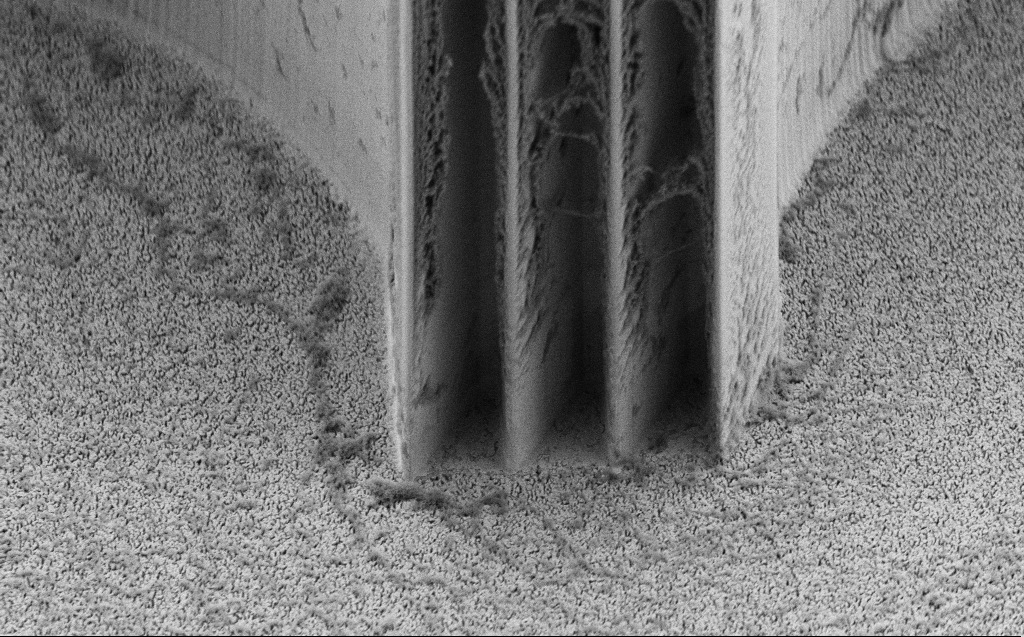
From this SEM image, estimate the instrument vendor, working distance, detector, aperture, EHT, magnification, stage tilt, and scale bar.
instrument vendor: Zeiss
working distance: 7 mm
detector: SE2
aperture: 30 µm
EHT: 5 kV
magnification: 3.37 K X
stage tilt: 45°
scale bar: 10000 nm